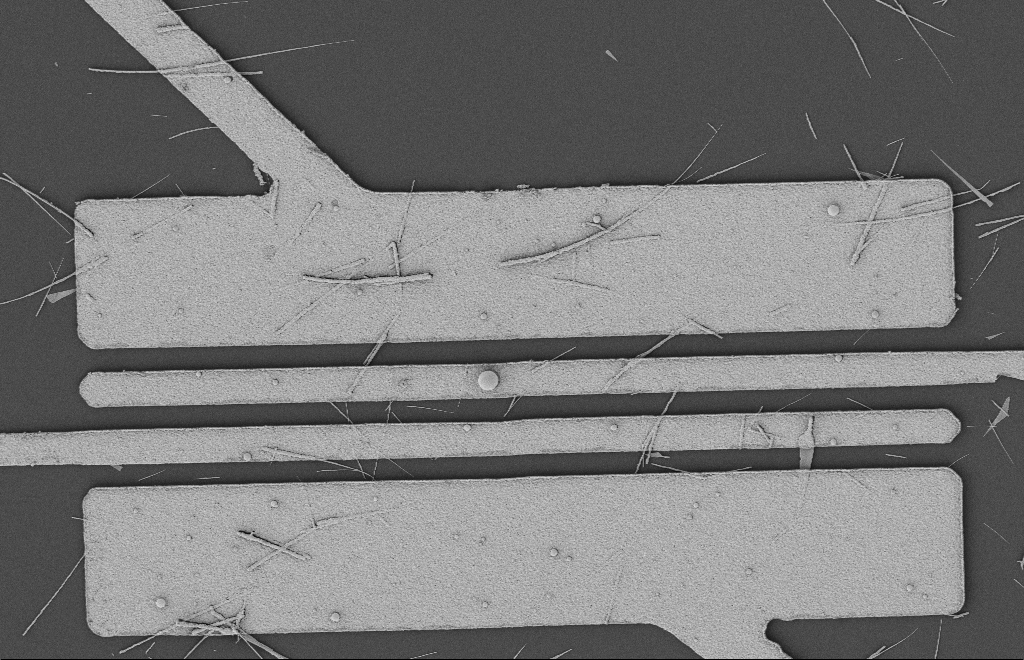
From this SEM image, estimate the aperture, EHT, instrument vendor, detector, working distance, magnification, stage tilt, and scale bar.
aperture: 20 µm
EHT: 2 kV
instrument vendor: Zeiss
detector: SE2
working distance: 12 mm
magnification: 5.26 K X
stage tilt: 0°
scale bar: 2000 nm